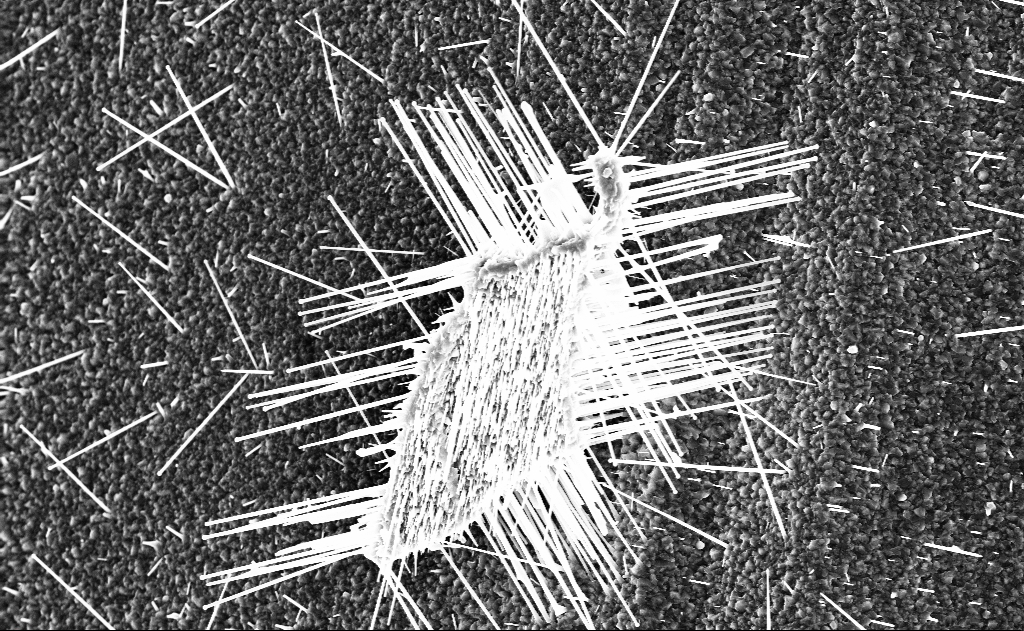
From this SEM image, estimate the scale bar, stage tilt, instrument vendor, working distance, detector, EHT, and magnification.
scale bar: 2000 nm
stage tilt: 0°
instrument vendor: Zeiss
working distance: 9 mm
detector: InLens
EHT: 10 kV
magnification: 10 K X